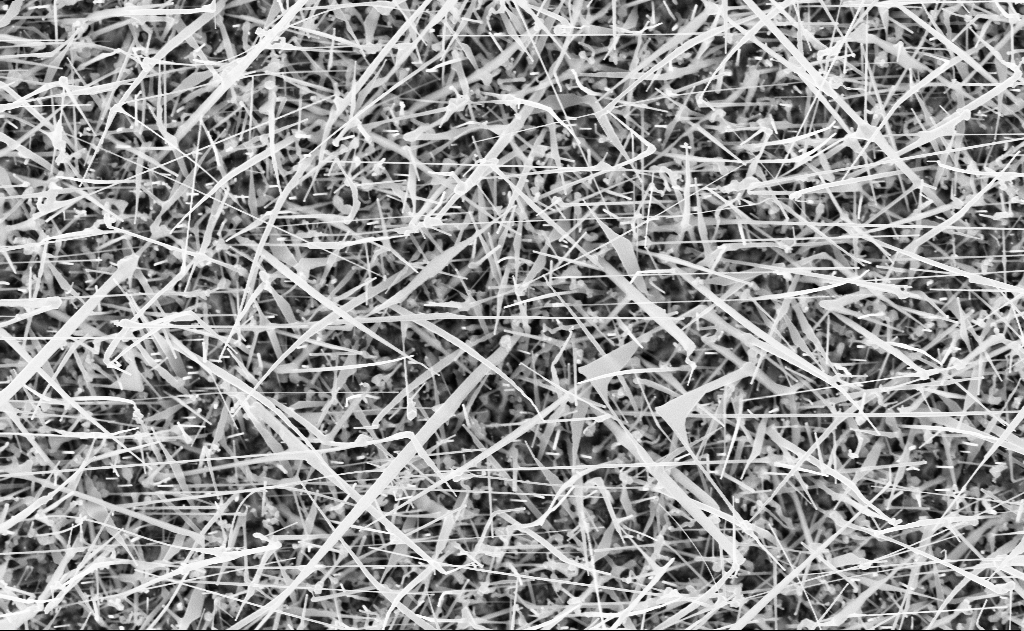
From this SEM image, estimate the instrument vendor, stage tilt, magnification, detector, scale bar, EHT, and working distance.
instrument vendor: Zeiss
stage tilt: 0°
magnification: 20 K X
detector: InLens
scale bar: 1000 nm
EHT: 10 kV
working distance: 11 mm